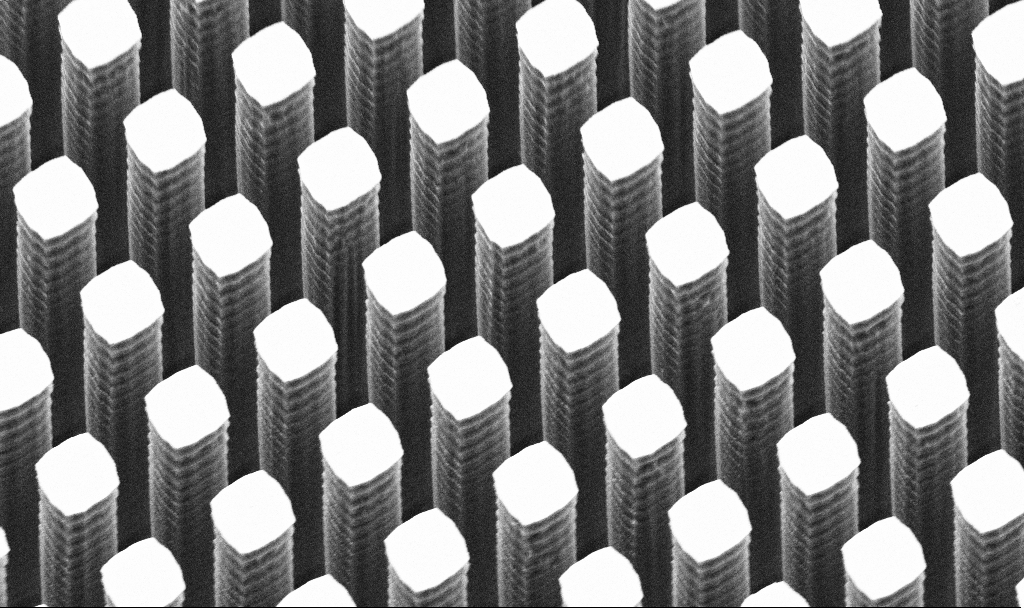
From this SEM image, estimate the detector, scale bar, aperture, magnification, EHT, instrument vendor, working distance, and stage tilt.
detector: SE2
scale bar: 2000 nm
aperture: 30 µm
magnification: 11.74 K X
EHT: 5 kV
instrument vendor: Zeiss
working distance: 7.5 mm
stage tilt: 45°